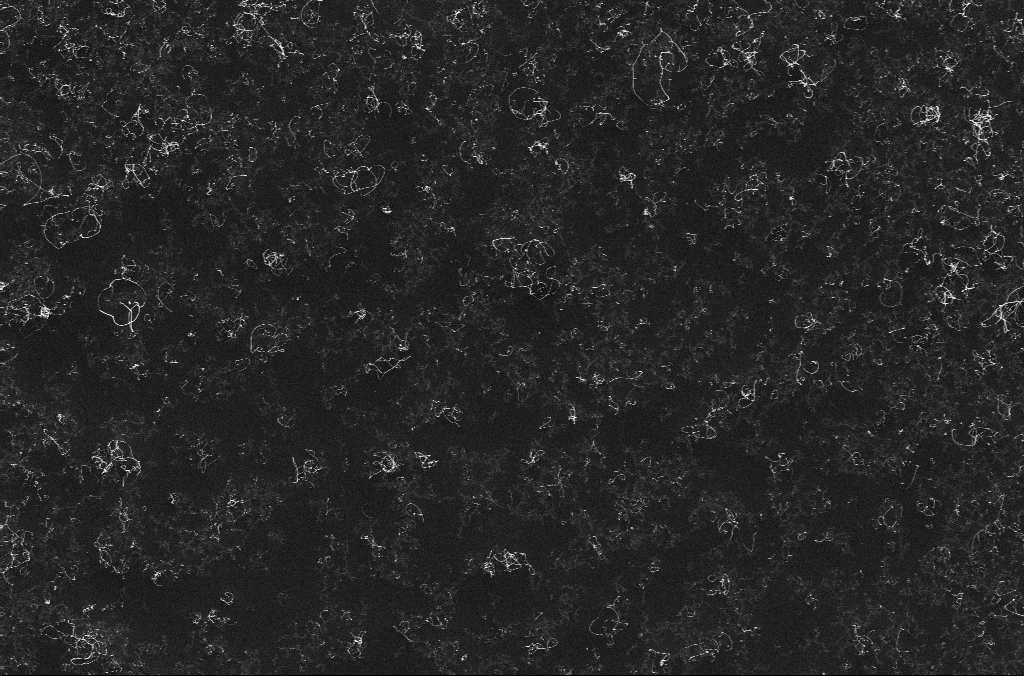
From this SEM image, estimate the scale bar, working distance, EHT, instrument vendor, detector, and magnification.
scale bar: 1000 nm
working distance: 3.2 mm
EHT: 10 kV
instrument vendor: Zeiss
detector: InLens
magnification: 15 K X